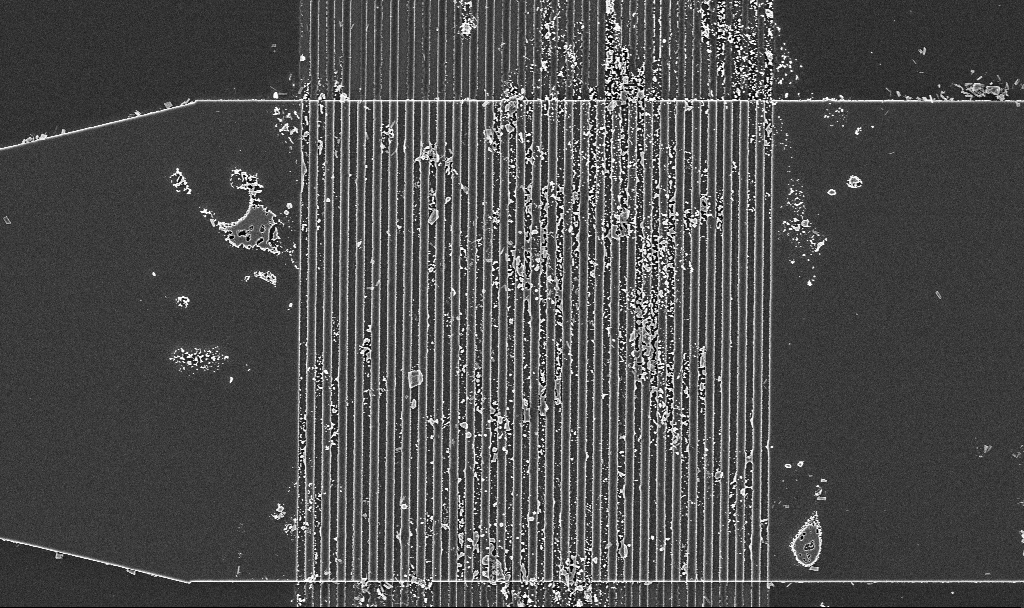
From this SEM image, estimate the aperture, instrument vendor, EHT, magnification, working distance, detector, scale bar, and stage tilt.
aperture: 30 µm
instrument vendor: Zeiss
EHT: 3 kV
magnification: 8.98 K X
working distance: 2.9 mm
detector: InLens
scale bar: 2000 nm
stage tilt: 0°